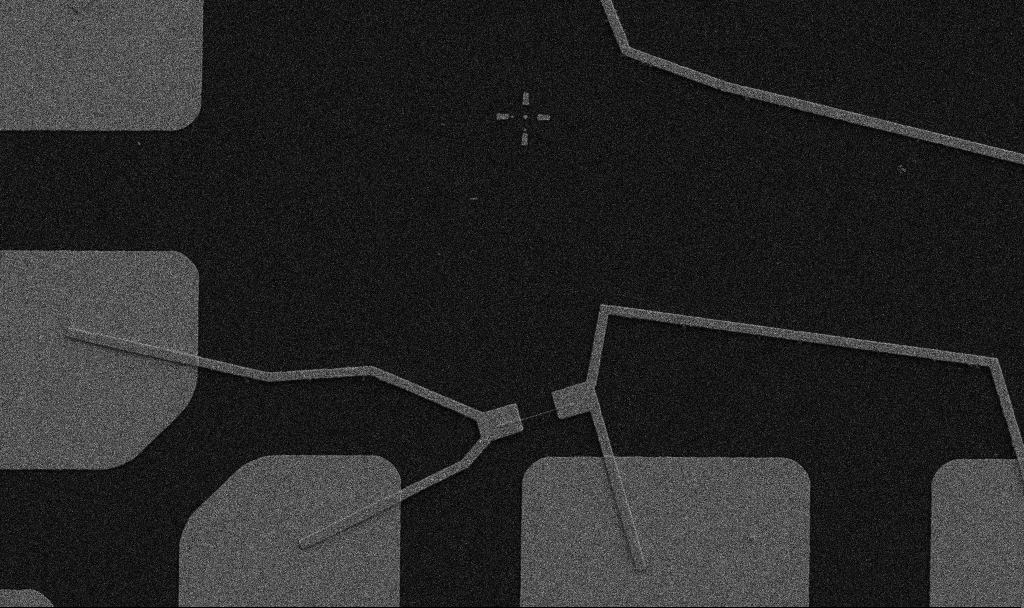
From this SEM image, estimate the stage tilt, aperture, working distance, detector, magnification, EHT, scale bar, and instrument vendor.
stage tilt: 0°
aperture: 30 µm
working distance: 10.7 mm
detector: SE2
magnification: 5 K X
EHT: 5 kV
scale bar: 10000 nm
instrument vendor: Zeiss